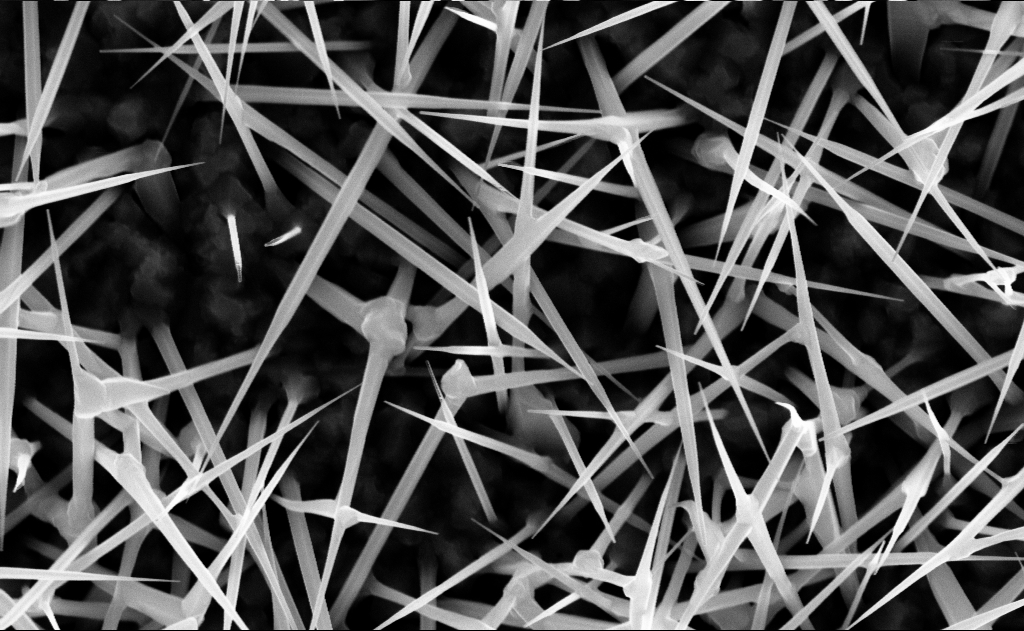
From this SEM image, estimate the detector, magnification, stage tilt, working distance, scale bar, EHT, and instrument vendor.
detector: InLens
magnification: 60 K X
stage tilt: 0°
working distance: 9 mm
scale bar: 1000 nm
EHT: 10 kV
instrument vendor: Zeiss